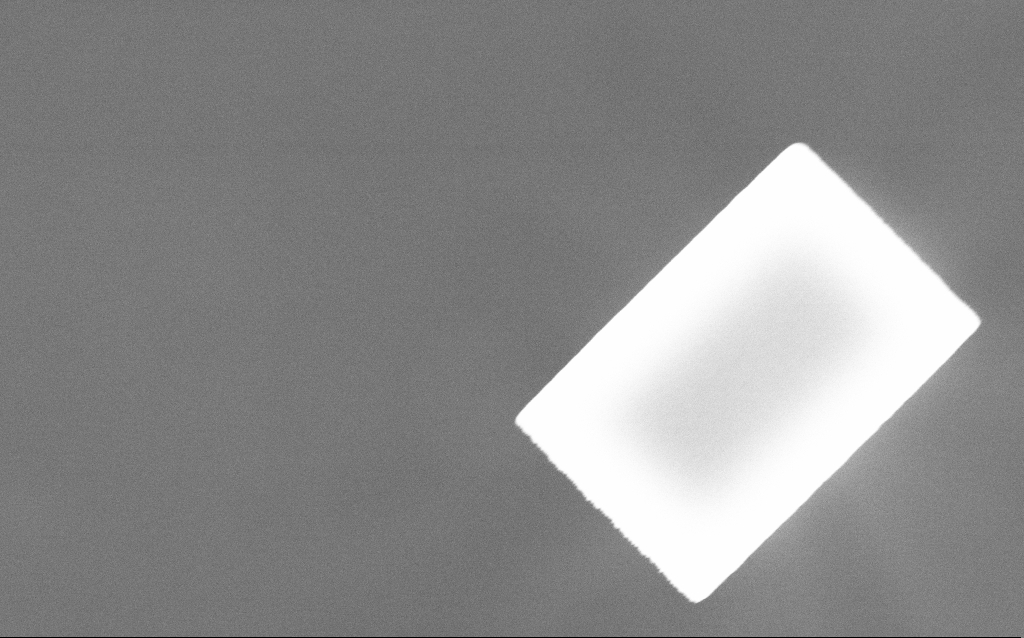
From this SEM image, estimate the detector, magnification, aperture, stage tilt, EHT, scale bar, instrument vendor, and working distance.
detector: InLens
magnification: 47.96 K X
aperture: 30 µm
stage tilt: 0°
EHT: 8 kV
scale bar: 1000 nm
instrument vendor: Zeiss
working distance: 7.1 mm